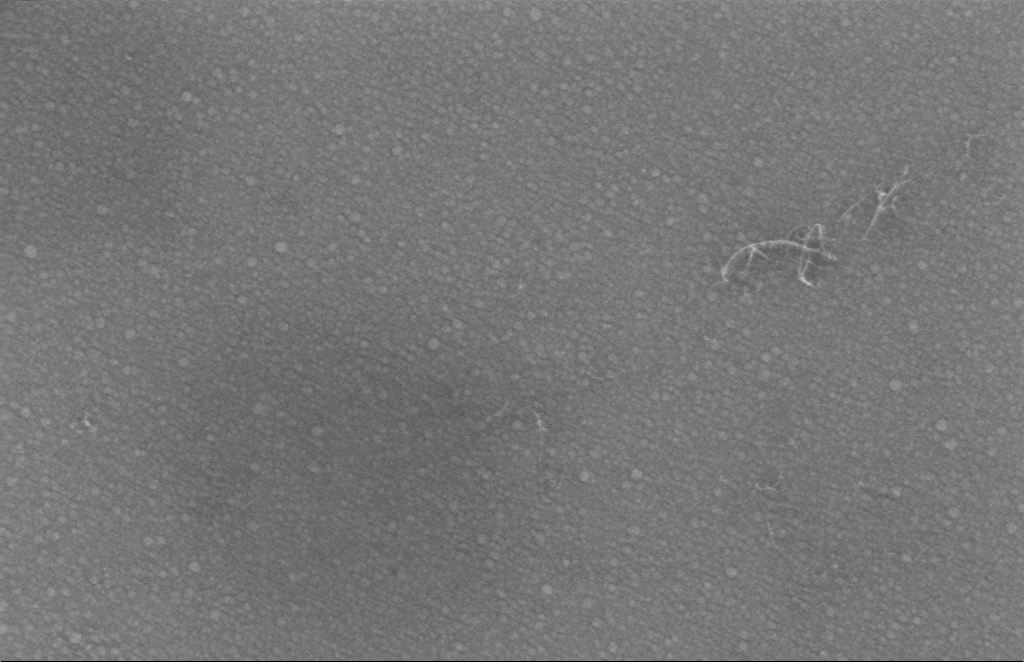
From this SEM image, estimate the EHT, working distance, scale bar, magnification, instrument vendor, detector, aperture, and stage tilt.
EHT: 5 kV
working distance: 5 mm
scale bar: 200 nm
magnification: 176.51 K X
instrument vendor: Zeiss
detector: InLens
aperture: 30 µm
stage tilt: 0°